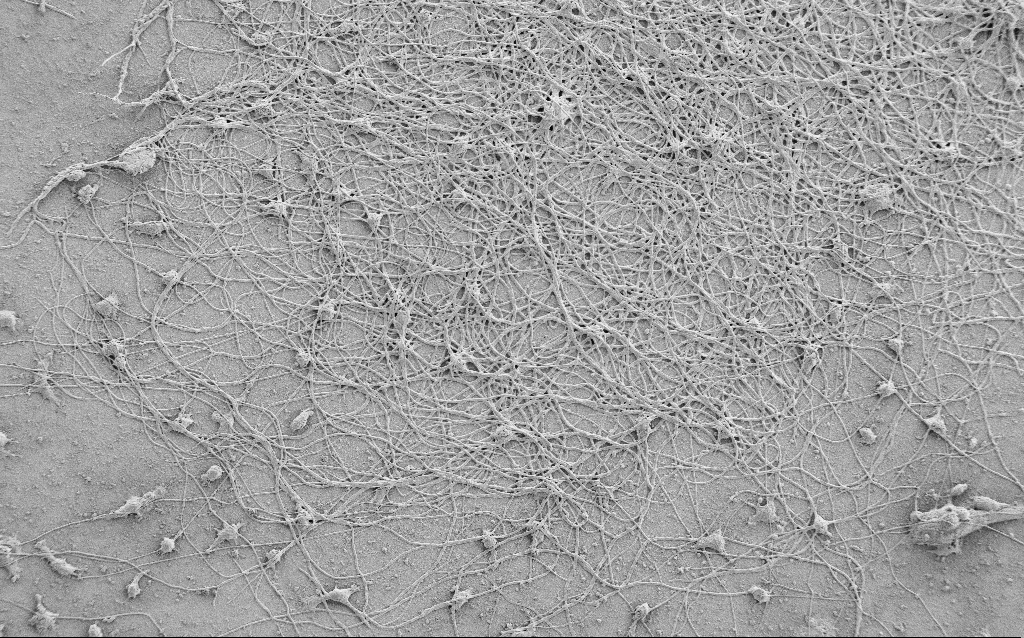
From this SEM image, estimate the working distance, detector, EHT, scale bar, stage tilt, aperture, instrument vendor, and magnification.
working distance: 6.8 mm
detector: SE2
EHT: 1.5 kV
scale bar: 100000 nm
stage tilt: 0°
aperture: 30 µm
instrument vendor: Zeiss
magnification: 0.5 K X